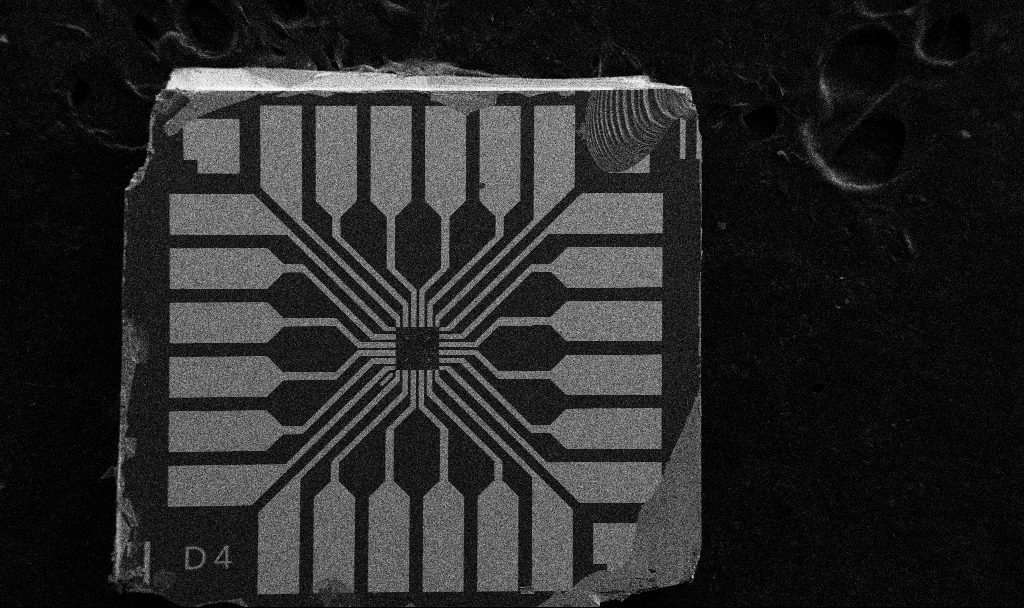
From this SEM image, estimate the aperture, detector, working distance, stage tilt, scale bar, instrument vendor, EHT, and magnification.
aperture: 30 µm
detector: SE2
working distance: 10.7 mm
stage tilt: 0°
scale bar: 200000 nm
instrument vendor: Zeiss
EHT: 5 kV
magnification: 0.1 K X